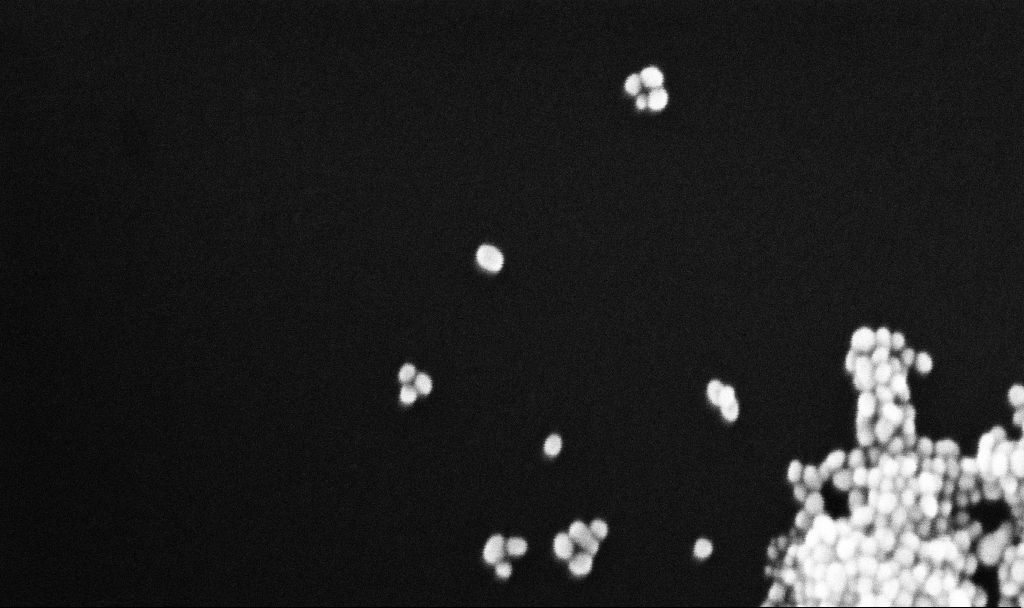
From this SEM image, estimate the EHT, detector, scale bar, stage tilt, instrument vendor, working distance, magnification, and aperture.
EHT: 10 kV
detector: InLens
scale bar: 100 nm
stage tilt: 0°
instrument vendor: Zeiss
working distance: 5.4 mm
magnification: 338.7 K X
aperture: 30 µm